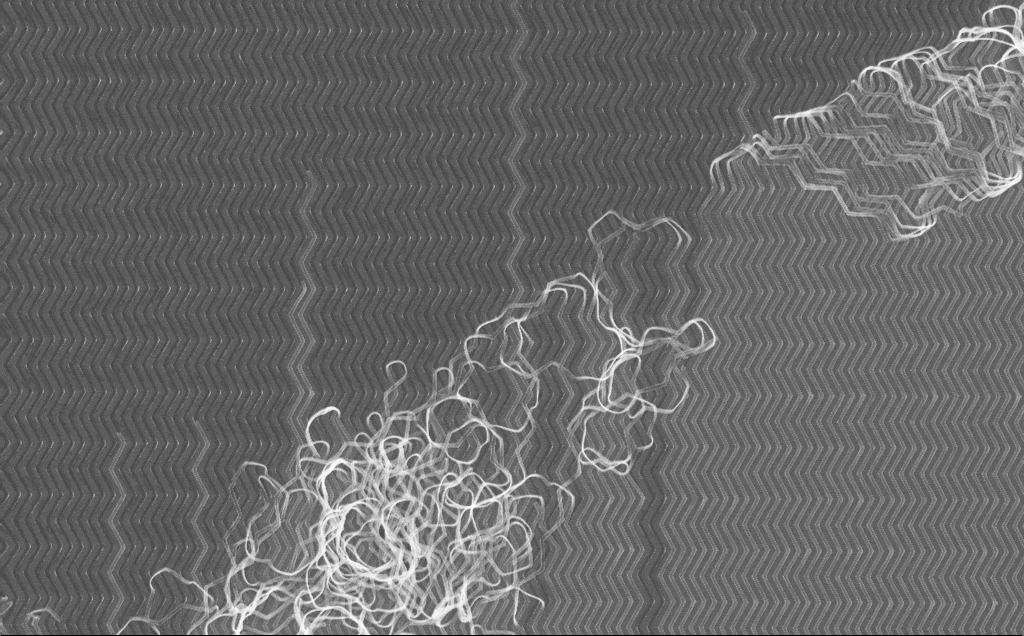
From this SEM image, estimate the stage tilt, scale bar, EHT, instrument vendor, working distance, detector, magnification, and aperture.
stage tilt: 0°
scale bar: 2000 nm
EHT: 10 kV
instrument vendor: Zeiss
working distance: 7 mm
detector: InLens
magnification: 9.59 K X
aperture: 30 µm